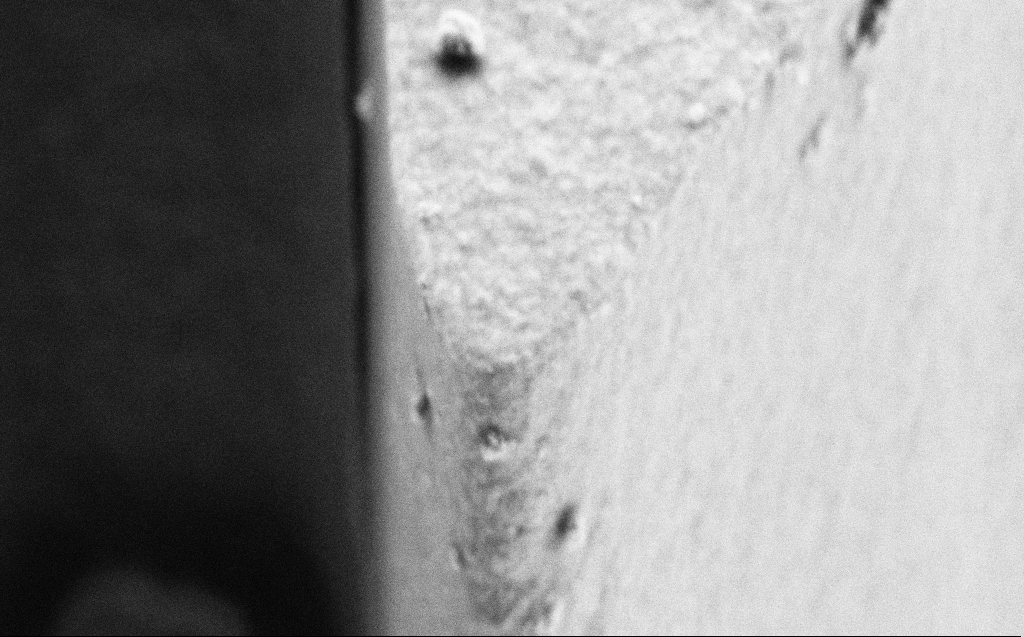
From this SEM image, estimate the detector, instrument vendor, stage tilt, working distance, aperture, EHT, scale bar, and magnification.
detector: SE2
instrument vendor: Zeiss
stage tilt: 45°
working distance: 4 mm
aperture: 30 µm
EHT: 3 kV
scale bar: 1000 nm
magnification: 57.32 K X